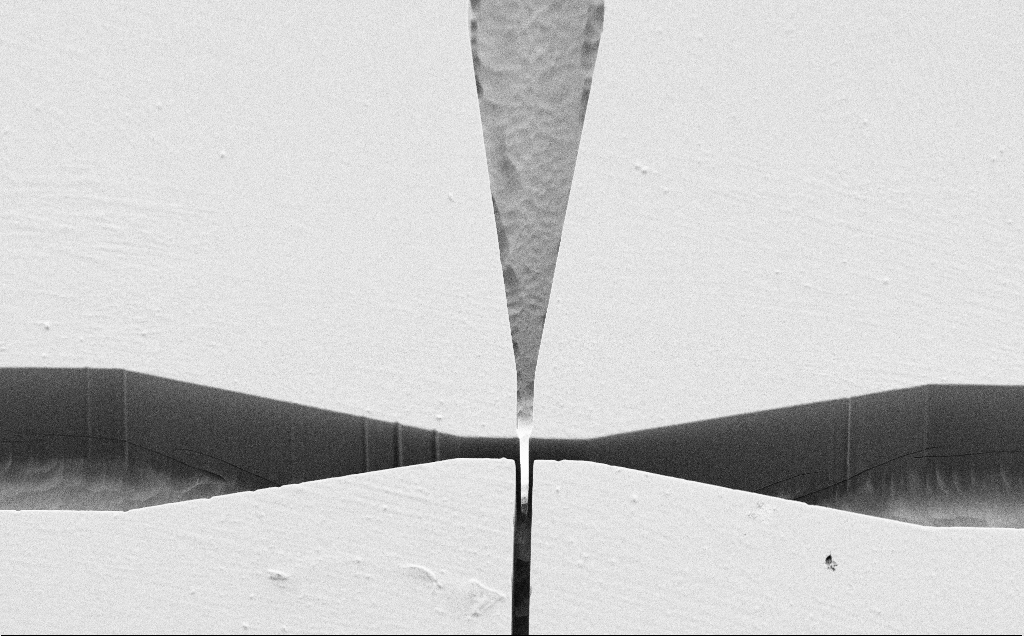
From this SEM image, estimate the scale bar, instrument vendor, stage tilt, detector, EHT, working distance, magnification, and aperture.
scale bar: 100000 nm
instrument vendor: Zeiss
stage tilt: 45°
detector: SE2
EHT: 1.2 kV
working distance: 5 mm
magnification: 0.617 K X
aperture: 30 µm